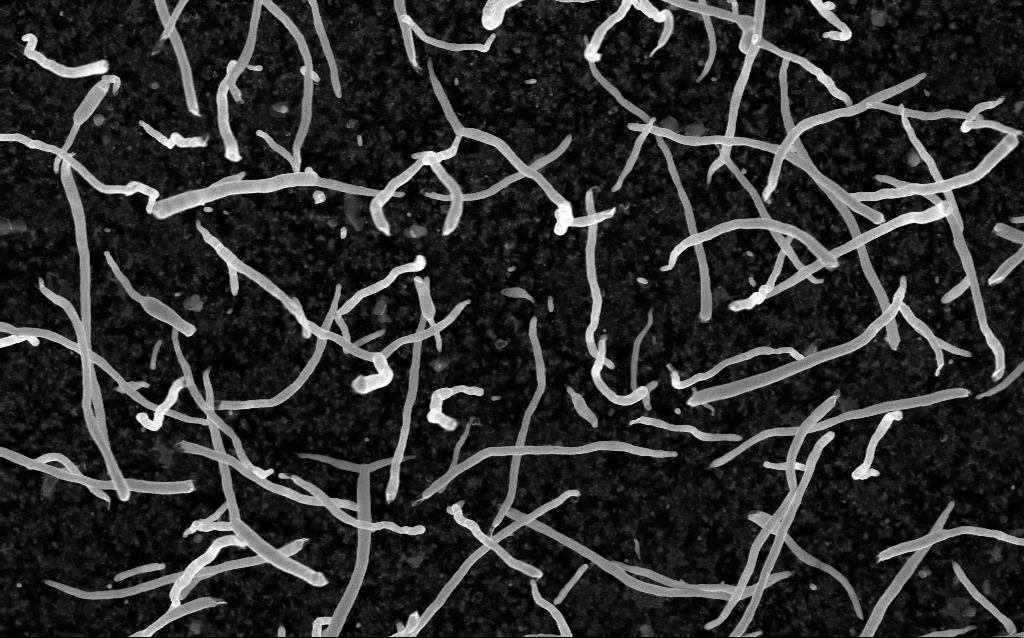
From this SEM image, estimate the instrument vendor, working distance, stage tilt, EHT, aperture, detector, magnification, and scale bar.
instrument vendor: Zeiss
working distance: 2.1 mm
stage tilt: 0°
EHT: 5 kV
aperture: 30 µm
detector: InLens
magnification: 50 K X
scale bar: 1000 nm